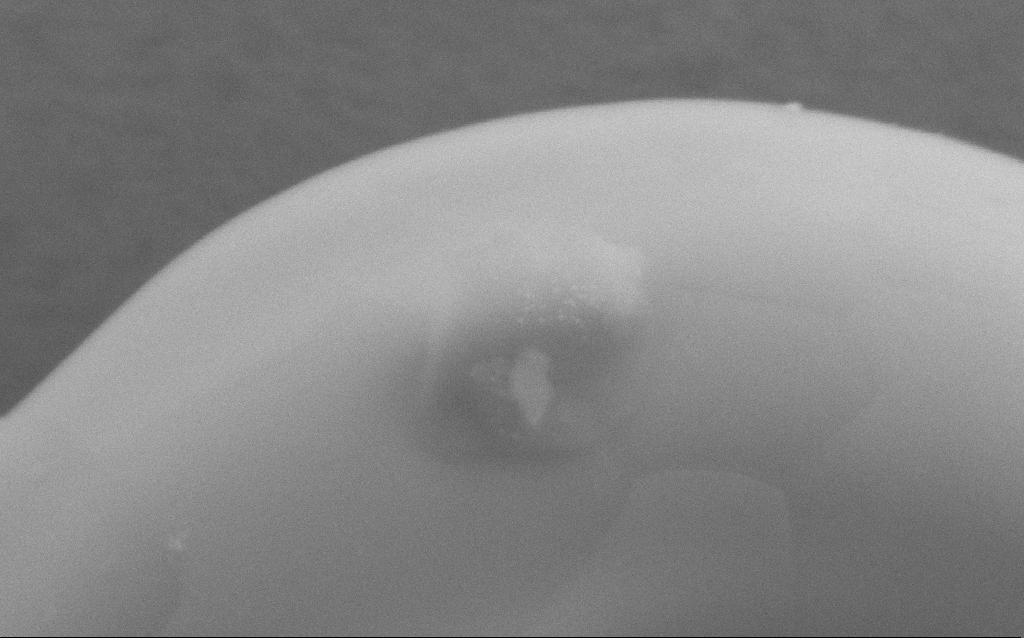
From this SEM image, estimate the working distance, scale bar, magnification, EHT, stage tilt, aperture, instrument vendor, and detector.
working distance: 4 mm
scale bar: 200 nm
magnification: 202.79 K X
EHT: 5 kV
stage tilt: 0°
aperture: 30 µm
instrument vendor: Zeiss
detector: SE2